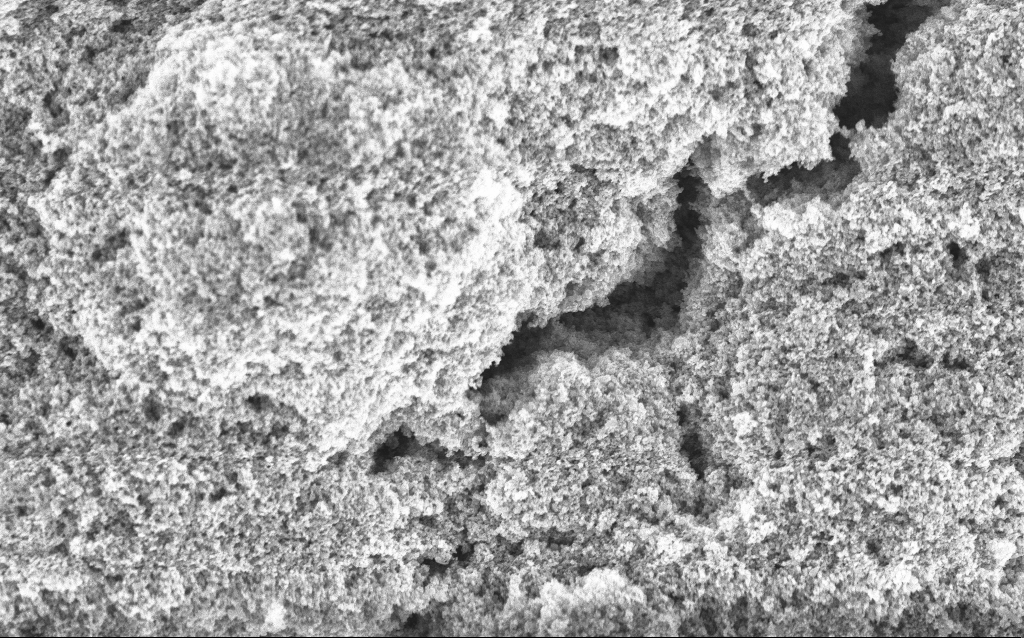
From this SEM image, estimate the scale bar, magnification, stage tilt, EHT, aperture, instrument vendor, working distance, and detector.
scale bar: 1000 nm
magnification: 37.88 K X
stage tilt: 0°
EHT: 5 kV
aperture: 30 µm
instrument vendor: Zeiss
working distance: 4.2 mm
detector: InLens